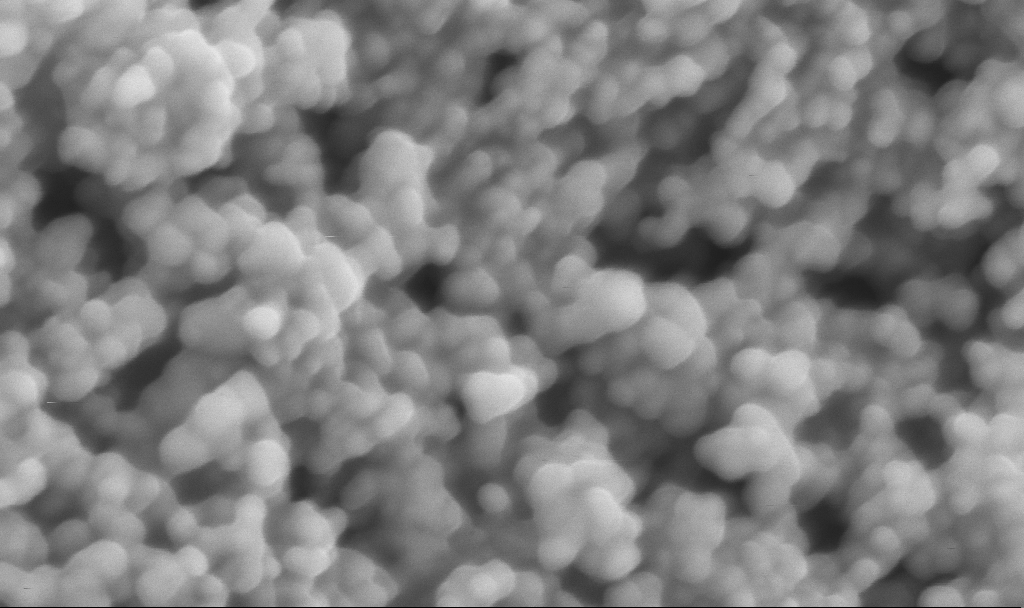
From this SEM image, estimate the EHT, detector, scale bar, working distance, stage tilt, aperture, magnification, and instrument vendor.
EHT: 3 kV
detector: InLens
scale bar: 200 nm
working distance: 2.5 mm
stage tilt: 0°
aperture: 30 µm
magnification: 325.68 K X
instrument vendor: Zeiss